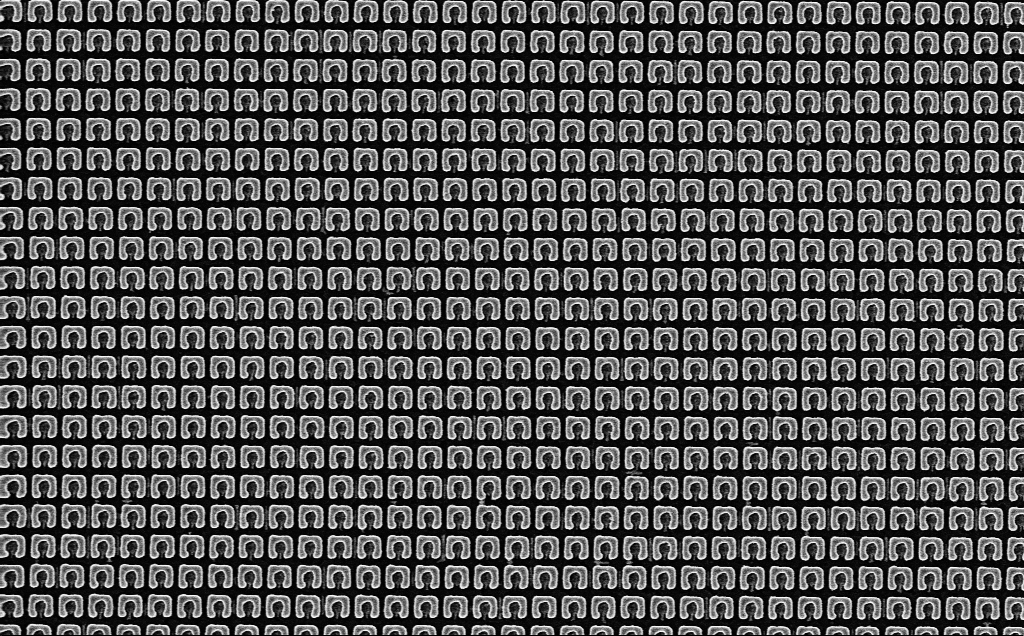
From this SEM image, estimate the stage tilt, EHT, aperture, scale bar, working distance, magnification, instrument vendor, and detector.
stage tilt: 0°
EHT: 5 kV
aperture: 30 µm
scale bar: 2000 nm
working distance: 2.6 mm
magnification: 23.65 K X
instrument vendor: Zeiss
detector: InLens